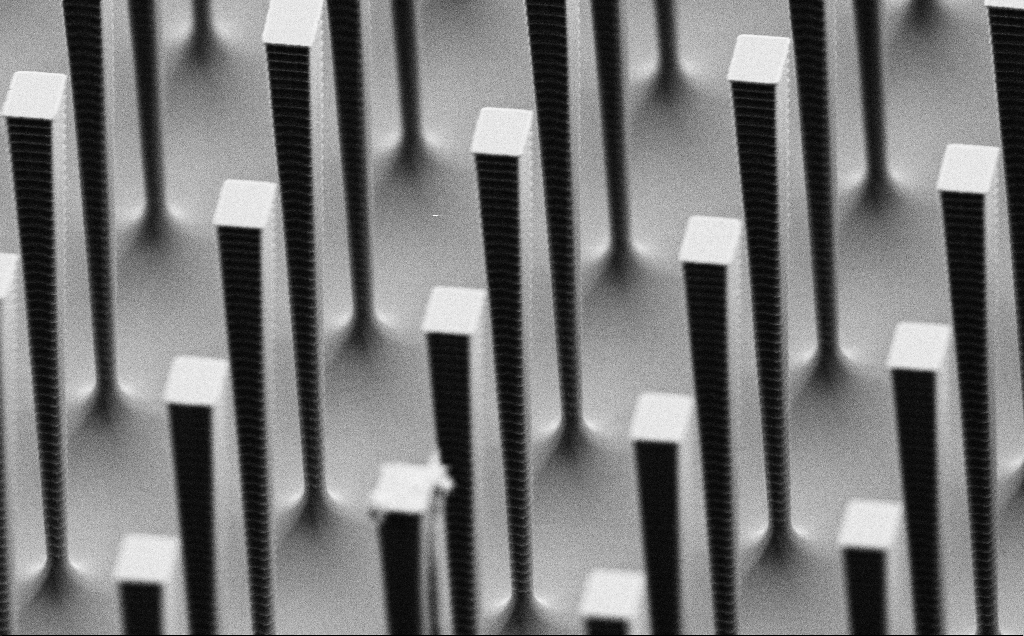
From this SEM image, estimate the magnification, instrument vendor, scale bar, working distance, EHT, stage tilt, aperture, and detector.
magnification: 9.57 K X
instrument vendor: Zeiss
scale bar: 2000 nm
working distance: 5.3 mm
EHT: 3 kV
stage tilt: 60°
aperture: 30 µm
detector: SE2